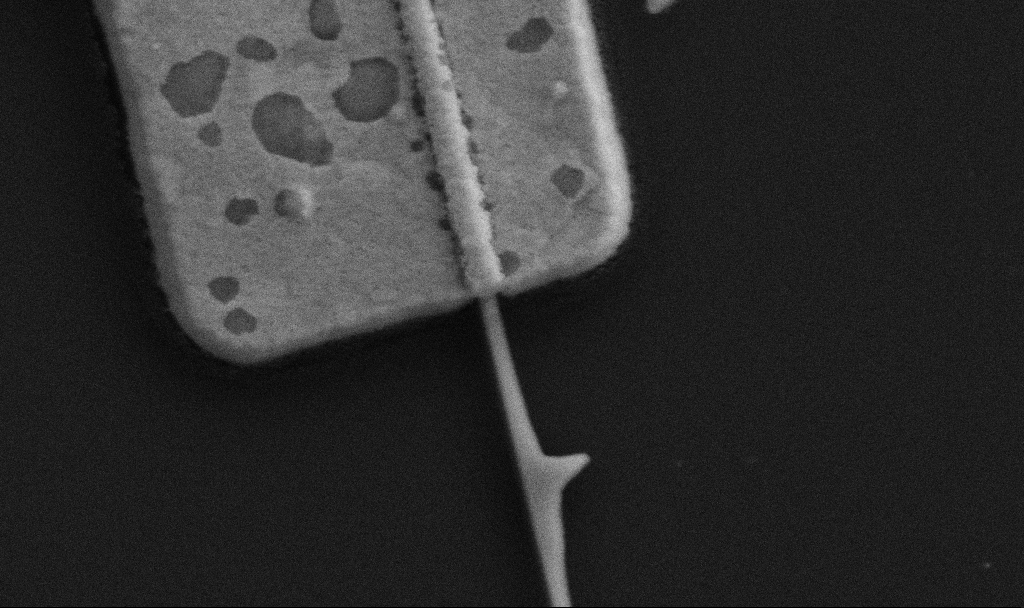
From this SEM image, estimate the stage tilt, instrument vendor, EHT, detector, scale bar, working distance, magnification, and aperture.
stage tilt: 0°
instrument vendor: Zeiss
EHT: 5 kV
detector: SE2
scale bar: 200 nm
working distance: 8.7 mm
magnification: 80 K X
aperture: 30 µm